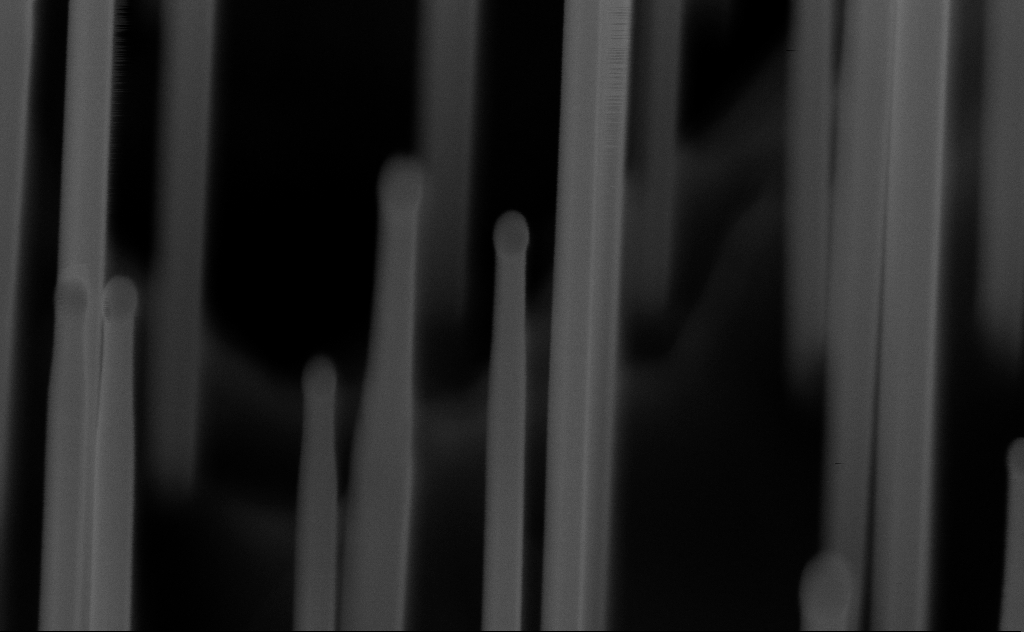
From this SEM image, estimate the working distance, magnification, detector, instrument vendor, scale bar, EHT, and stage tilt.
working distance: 6 mm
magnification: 221.61 K X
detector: InLens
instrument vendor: Zeiss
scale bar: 200 nm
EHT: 10 kV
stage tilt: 45°